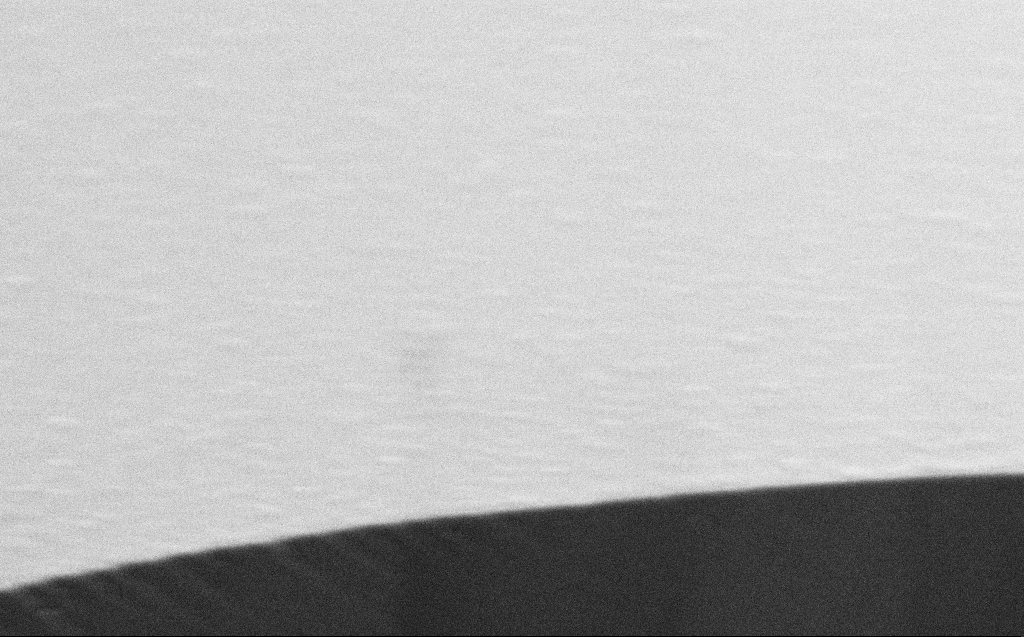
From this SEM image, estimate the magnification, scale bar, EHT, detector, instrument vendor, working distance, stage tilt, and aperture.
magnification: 62.3 K X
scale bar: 1000 nm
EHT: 2 kV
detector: SE2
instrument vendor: Zeiss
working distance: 3 mm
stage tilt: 45°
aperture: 30 µm